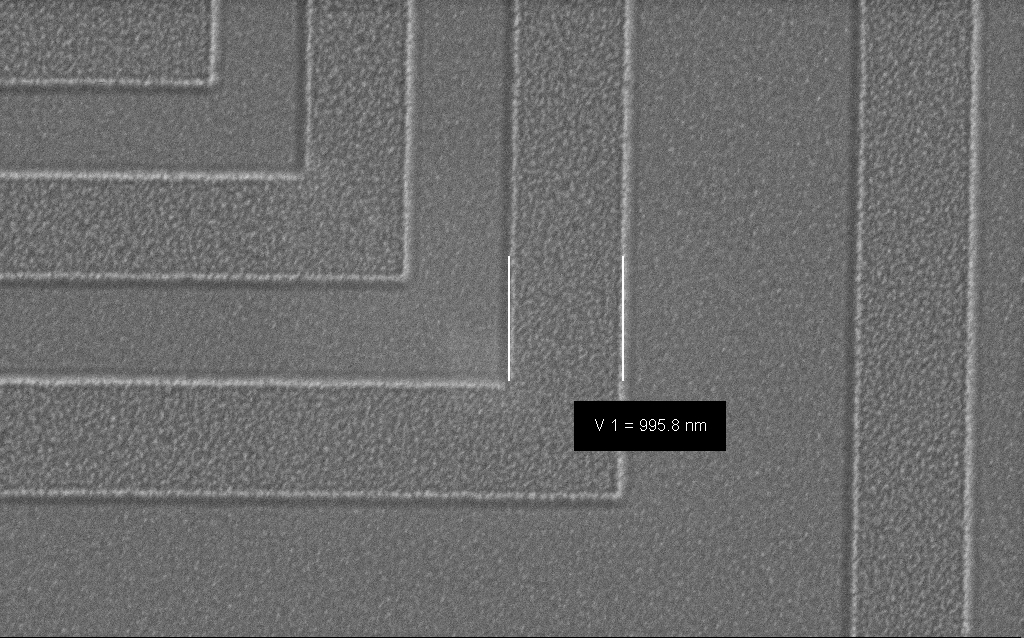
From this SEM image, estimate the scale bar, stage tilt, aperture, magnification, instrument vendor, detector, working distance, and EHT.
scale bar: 1000 nm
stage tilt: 0°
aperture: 30 µm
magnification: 42.04 K X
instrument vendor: Zeiss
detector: SE2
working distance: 6 mm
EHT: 1.5 kV